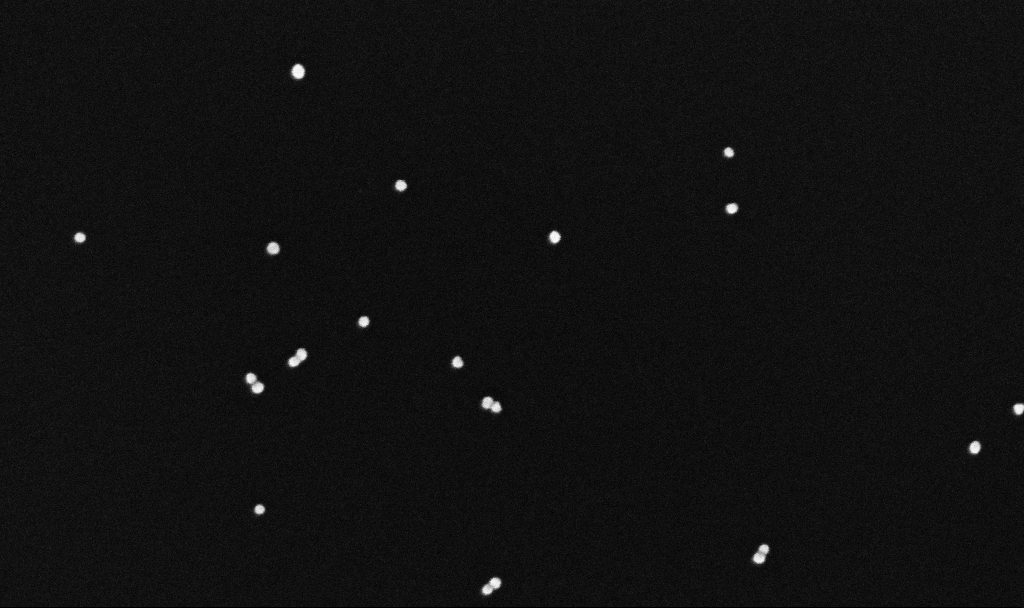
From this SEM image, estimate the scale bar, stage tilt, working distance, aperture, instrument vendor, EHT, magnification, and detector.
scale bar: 100 nm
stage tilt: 0°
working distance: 3.4 mm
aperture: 30 µm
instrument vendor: Zeiss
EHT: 10 kV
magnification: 300 K X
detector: InLens